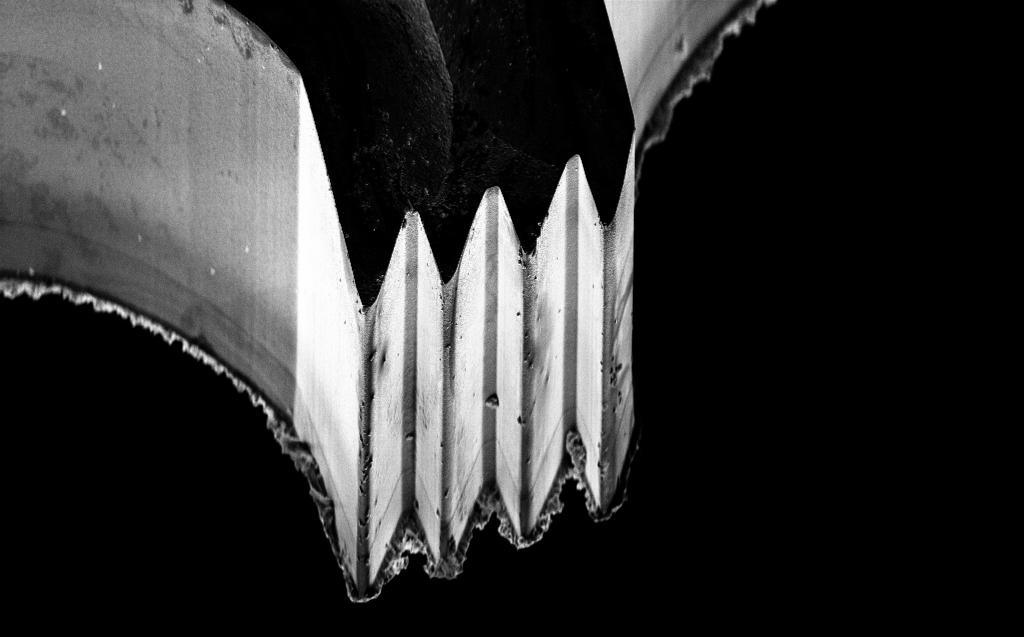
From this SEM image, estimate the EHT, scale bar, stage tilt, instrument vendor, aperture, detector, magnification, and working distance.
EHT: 5 kV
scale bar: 10000 nm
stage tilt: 45°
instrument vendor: Zeiss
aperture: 30 µm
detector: InLens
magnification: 2.77 K X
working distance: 6 mm